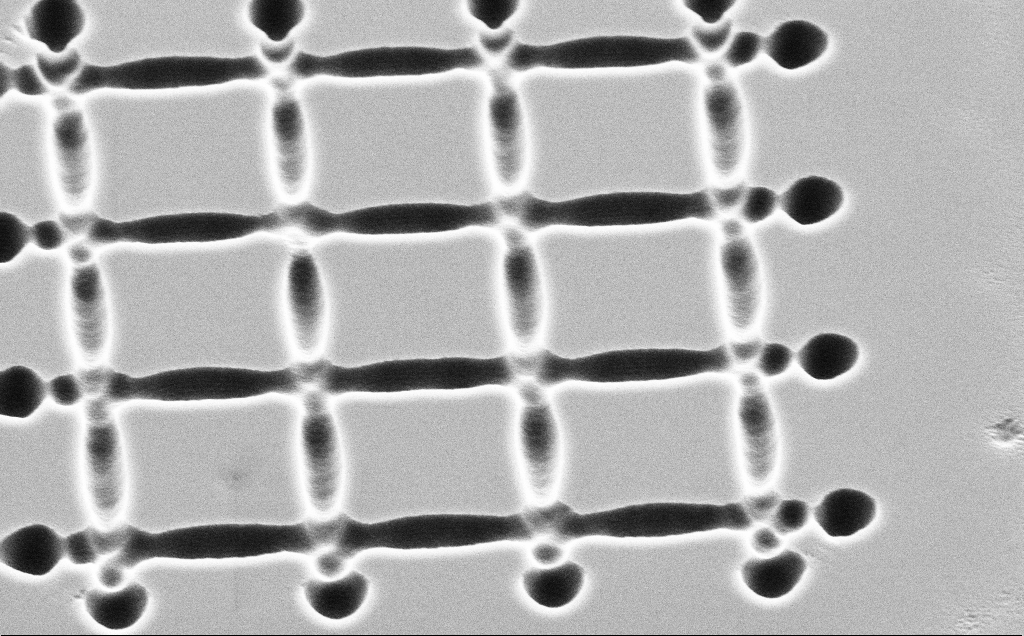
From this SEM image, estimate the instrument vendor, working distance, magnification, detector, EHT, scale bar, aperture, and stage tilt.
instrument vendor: Zeiss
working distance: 12 mm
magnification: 12.46 K X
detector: SE2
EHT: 5 kV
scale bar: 2000 nm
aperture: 30 µm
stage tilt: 45°